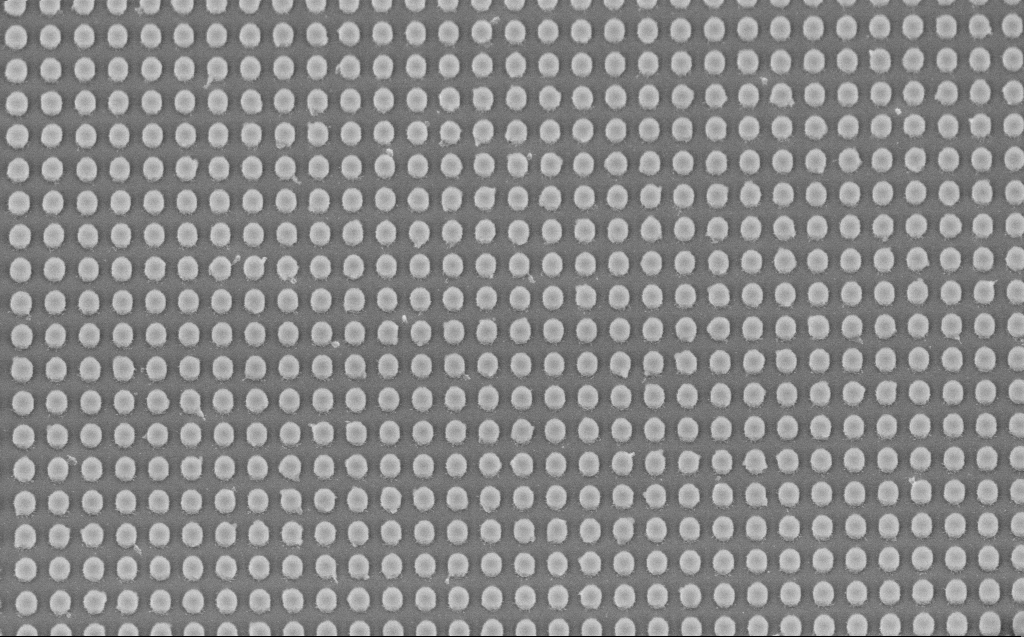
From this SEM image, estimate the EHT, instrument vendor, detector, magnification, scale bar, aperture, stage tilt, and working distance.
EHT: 3 kV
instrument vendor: Zeiss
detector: InLens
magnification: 30.59 K X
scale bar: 1000 nm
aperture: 30 µm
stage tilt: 30°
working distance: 6 mm